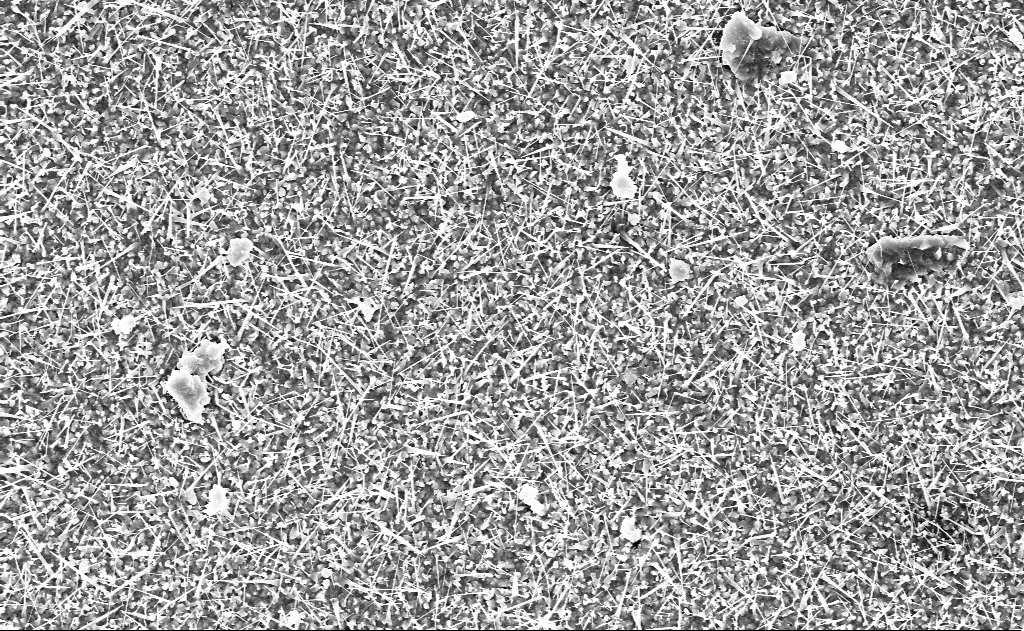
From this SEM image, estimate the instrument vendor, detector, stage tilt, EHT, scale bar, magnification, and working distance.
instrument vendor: Zeiss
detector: InLens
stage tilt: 0°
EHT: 10 kV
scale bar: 2000 nm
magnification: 10 K X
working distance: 12 mm